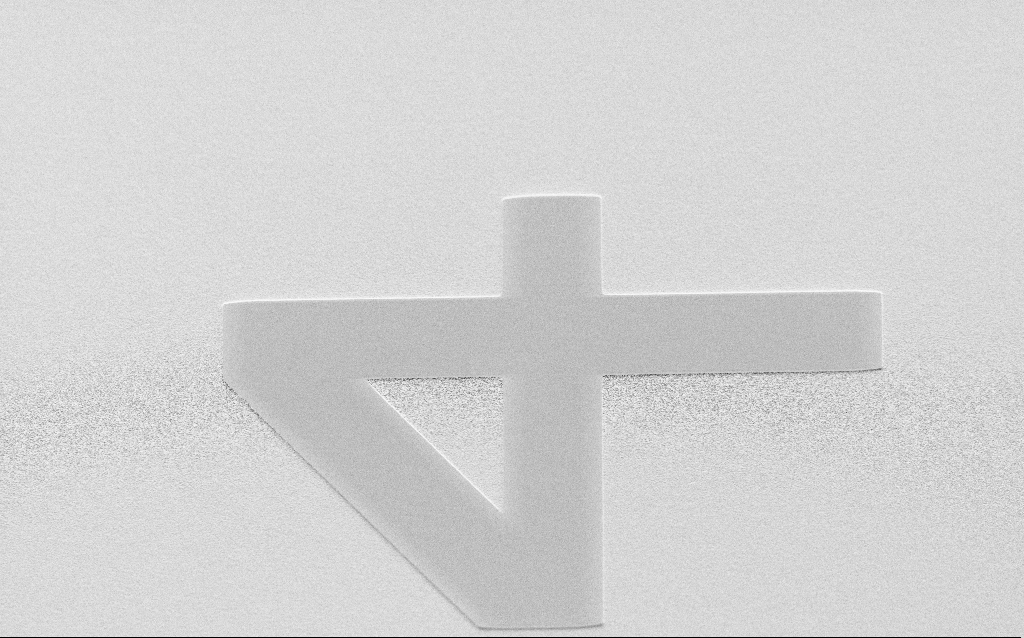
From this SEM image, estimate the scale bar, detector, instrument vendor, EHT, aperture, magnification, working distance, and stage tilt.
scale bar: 20000 nm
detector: SE2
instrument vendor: Zeiss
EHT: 3 kV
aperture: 30 µm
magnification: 0.937 K X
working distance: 8 mm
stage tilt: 45°